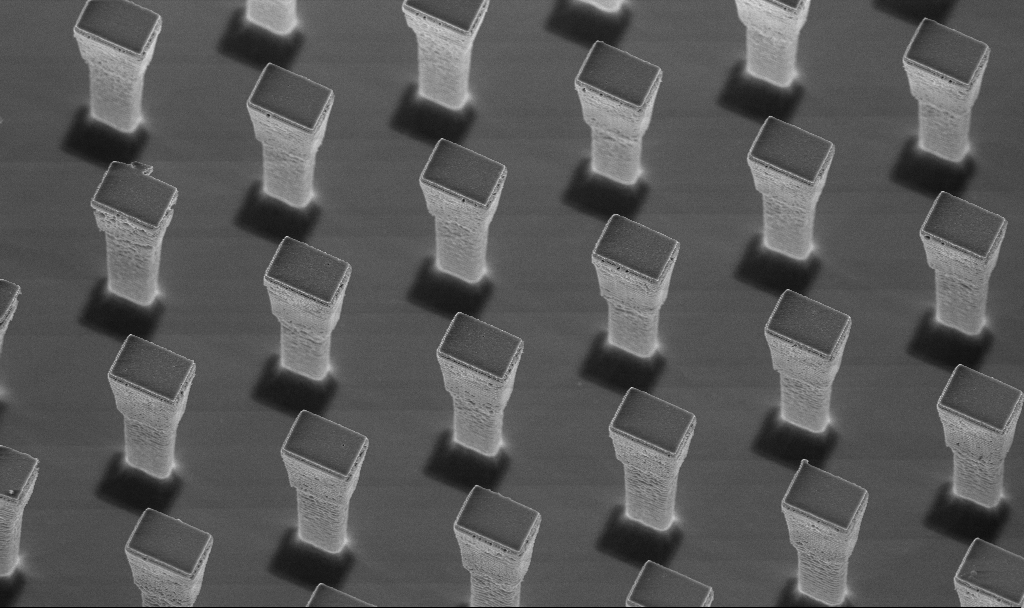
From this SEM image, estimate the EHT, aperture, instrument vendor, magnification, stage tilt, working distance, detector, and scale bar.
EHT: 5 kV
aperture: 30 µm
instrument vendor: Zeiss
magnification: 5.85 K X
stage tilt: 20°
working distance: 4.2 mm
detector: InLens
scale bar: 10000 nm